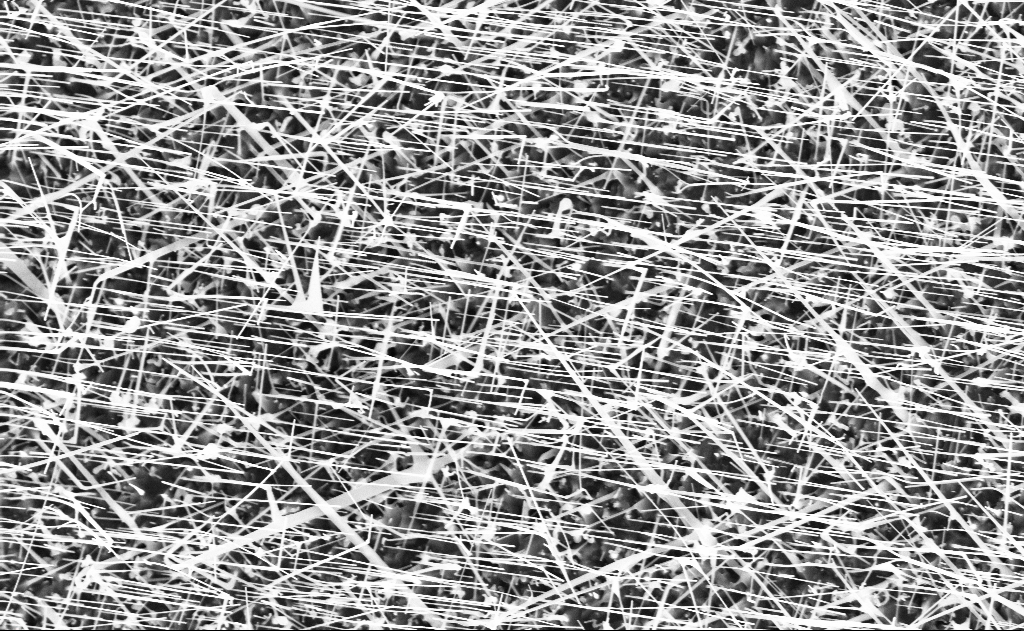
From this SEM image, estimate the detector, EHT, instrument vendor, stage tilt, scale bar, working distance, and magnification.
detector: InLens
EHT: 10 kV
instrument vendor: Zeiss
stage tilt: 0°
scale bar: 2000 nm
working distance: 10 mm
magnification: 20 K X